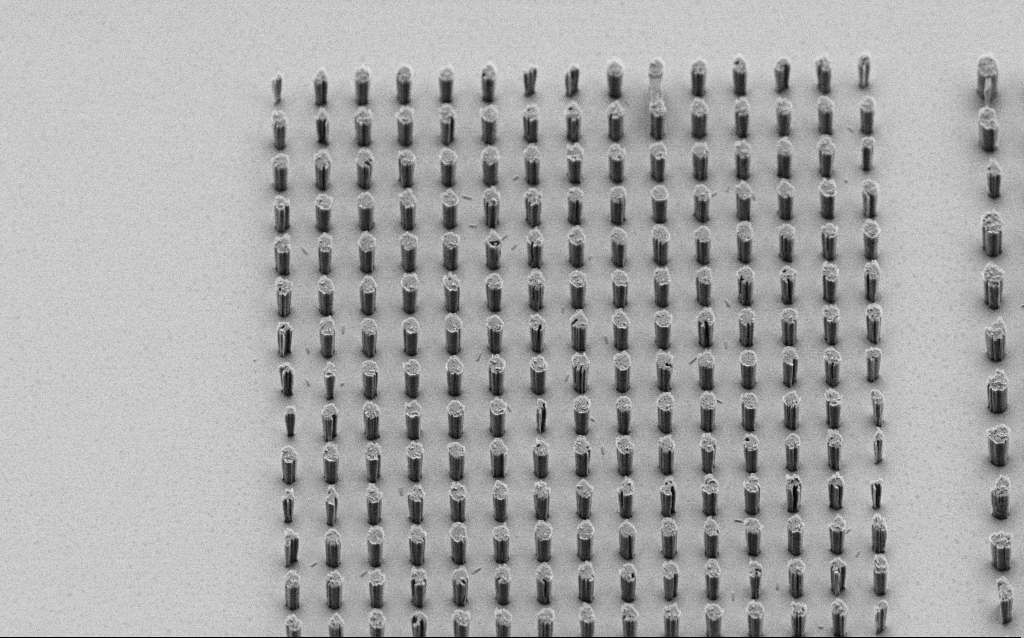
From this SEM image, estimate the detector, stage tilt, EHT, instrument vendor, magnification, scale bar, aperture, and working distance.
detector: SE2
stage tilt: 45°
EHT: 2 kV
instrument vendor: Zeiss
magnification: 9.54 K X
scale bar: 2000 nm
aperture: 30 µm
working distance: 7 mm